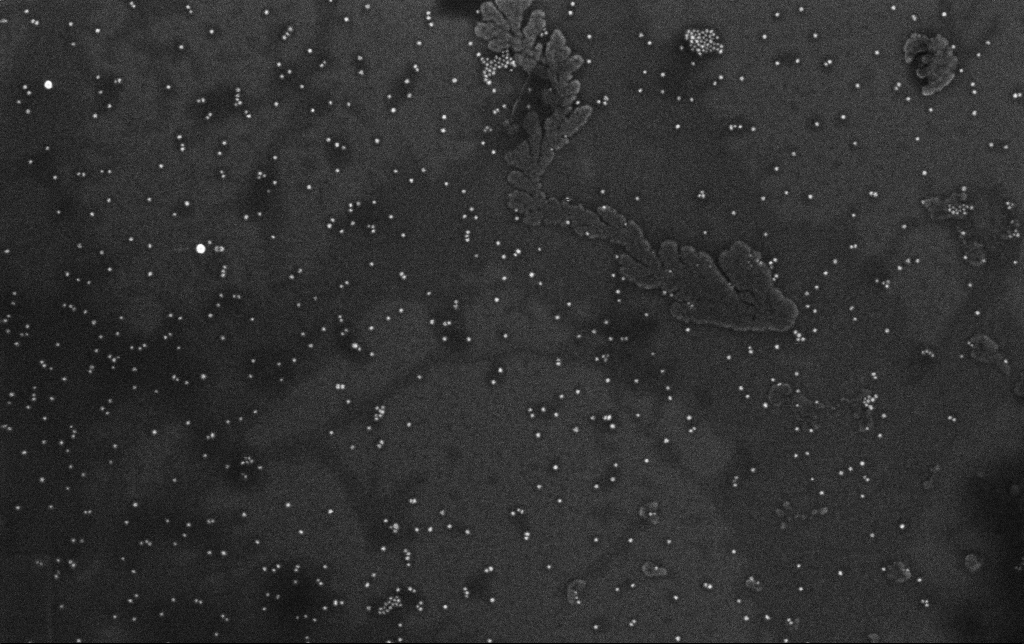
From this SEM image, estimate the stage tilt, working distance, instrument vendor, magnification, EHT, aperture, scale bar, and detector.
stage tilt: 0°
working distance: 3.2 mm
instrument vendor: Zeiss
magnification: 100 K X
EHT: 10 kV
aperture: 30 µm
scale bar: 200 nm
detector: InLens